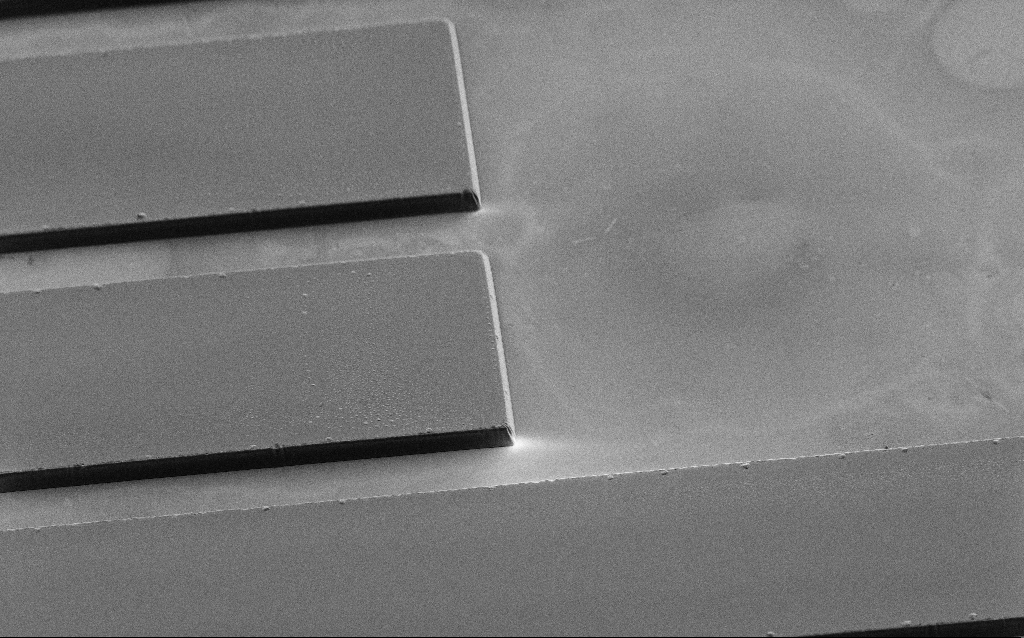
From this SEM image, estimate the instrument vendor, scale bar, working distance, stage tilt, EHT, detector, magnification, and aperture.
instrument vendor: Zeiss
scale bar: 20000 nm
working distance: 6 mm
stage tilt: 45°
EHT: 1.2 kV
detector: SE2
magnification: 1.16 K X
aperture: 30 µm